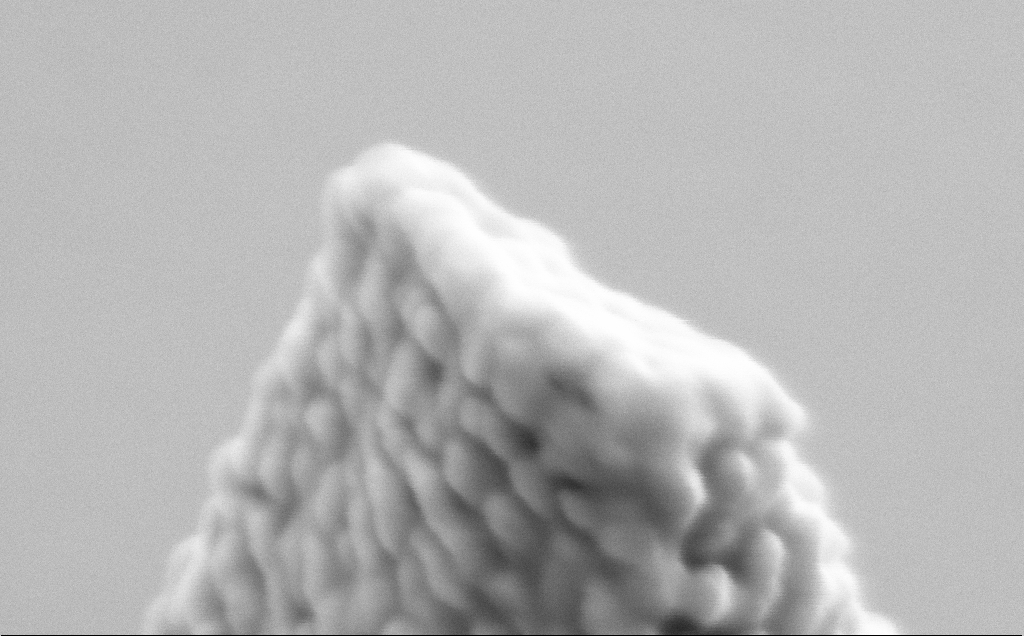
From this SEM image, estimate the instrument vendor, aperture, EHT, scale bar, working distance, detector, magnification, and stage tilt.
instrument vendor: Zeiss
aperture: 30 µm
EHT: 5 kV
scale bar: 100 nm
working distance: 10 mm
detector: SE2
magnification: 464.4 K X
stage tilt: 45.6°